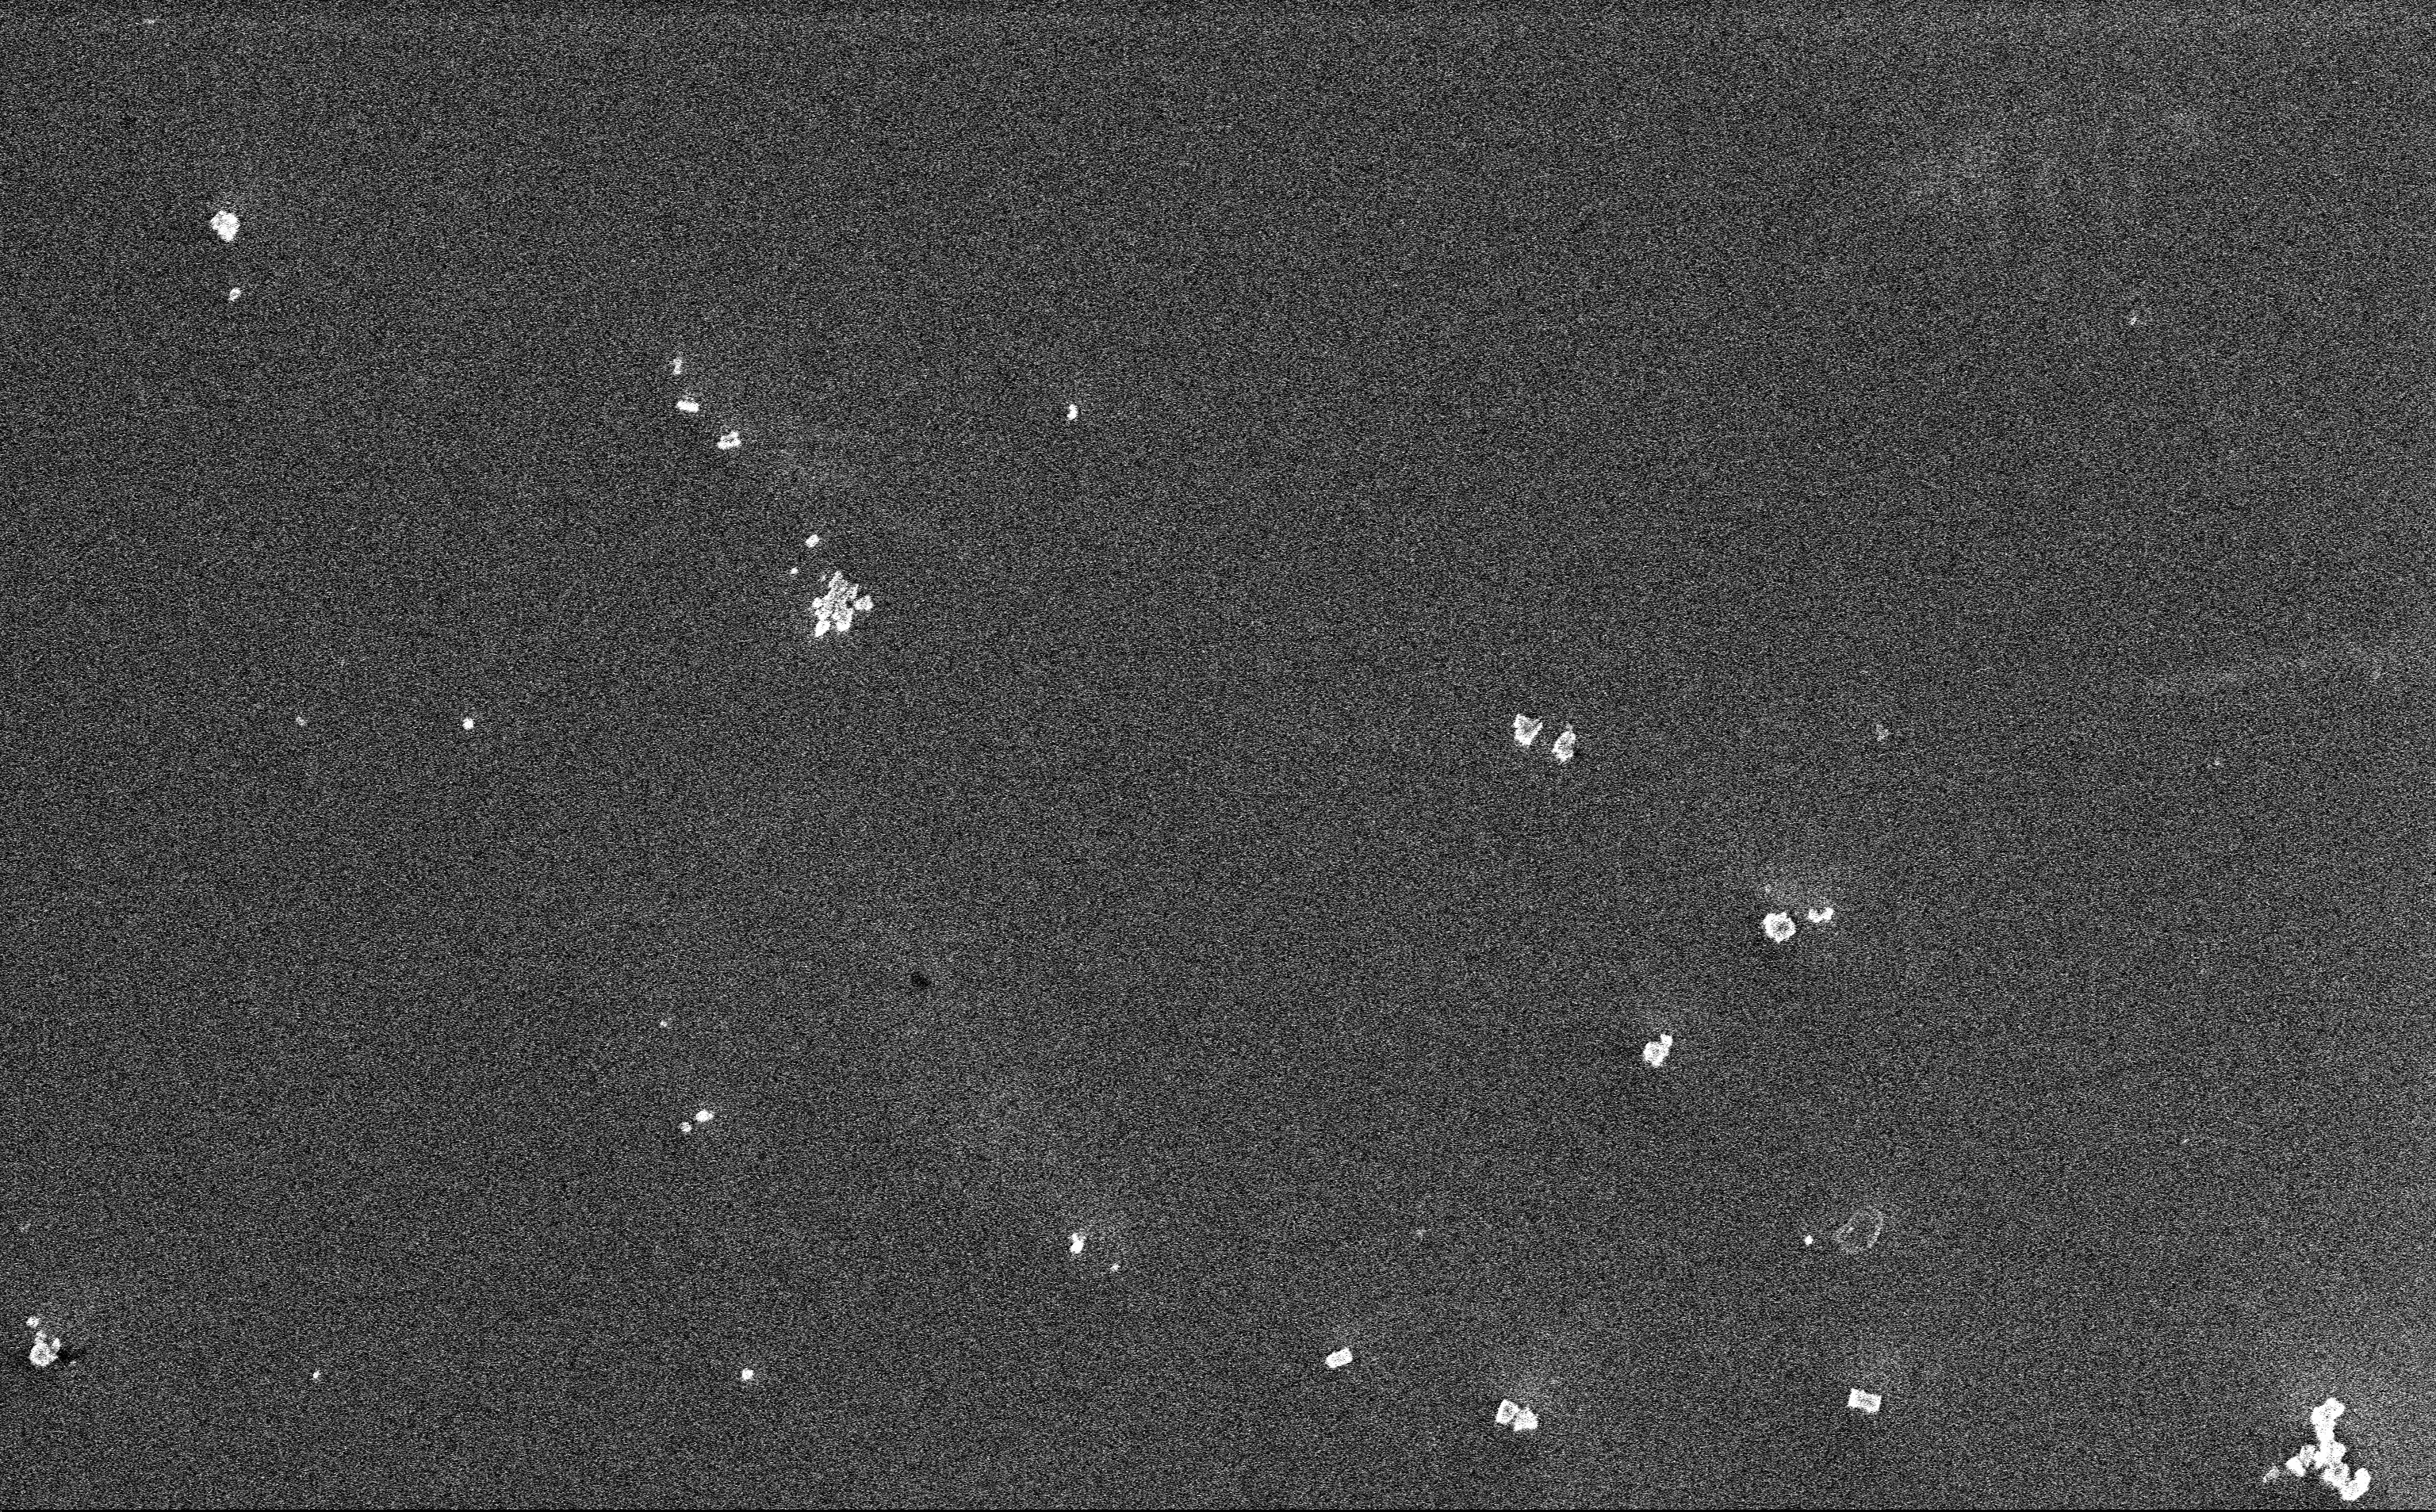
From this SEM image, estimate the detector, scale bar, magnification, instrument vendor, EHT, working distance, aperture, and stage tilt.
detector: InLens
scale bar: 2000 nm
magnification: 12.85 K X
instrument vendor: Zeiss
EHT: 3 kV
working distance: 3 mm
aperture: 30 µm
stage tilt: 0°